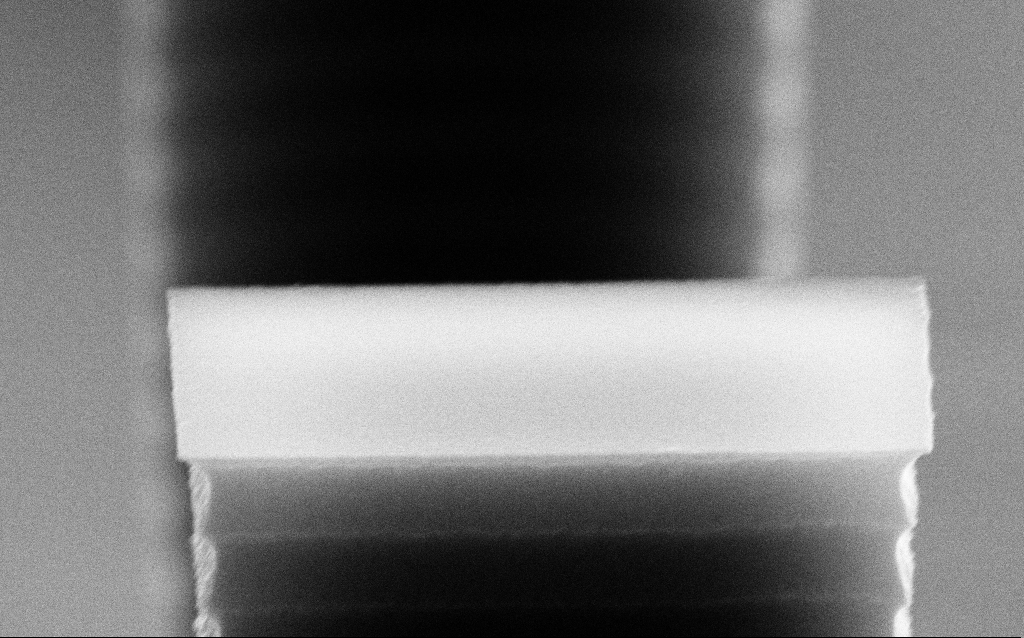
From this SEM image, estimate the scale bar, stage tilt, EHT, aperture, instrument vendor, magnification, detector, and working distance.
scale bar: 200 nm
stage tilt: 70°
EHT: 10 kV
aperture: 30 µm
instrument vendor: Zeiss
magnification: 94.17 K X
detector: SE2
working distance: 7.5 mm